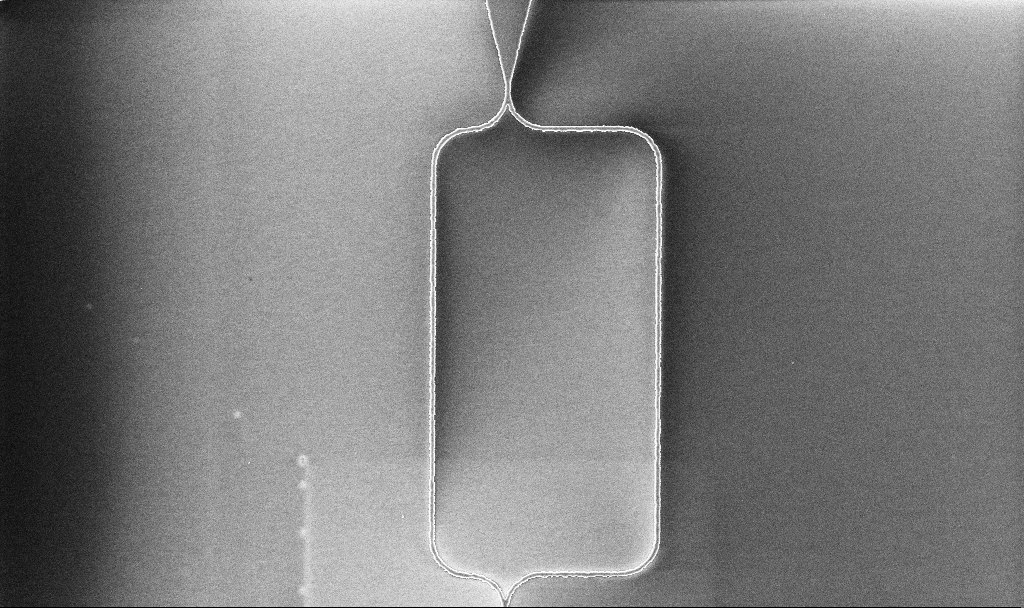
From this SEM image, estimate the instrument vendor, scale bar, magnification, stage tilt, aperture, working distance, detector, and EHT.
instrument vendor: Zeiss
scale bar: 10000 nm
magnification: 2.88 K X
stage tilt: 0°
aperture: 30 µm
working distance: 10.1 mm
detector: InLens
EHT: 5 kV